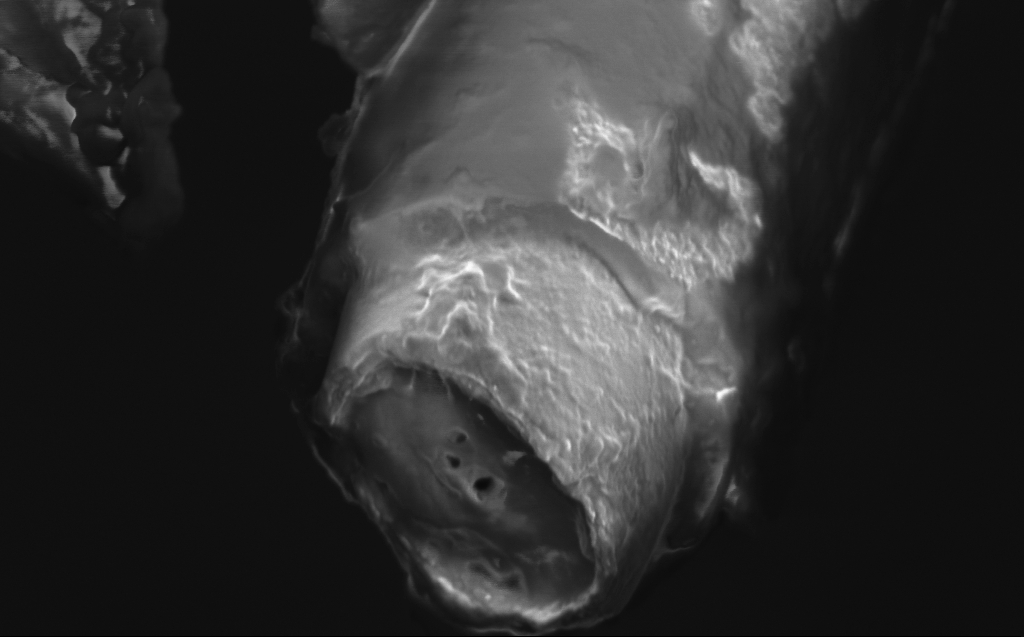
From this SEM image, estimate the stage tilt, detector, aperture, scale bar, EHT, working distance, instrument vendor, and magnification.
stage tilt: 45°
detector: InLens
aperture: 30 µm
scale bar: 1000 nm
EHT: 1 kV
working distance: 4 mm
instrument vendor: Zeiss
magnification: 51.78 K X